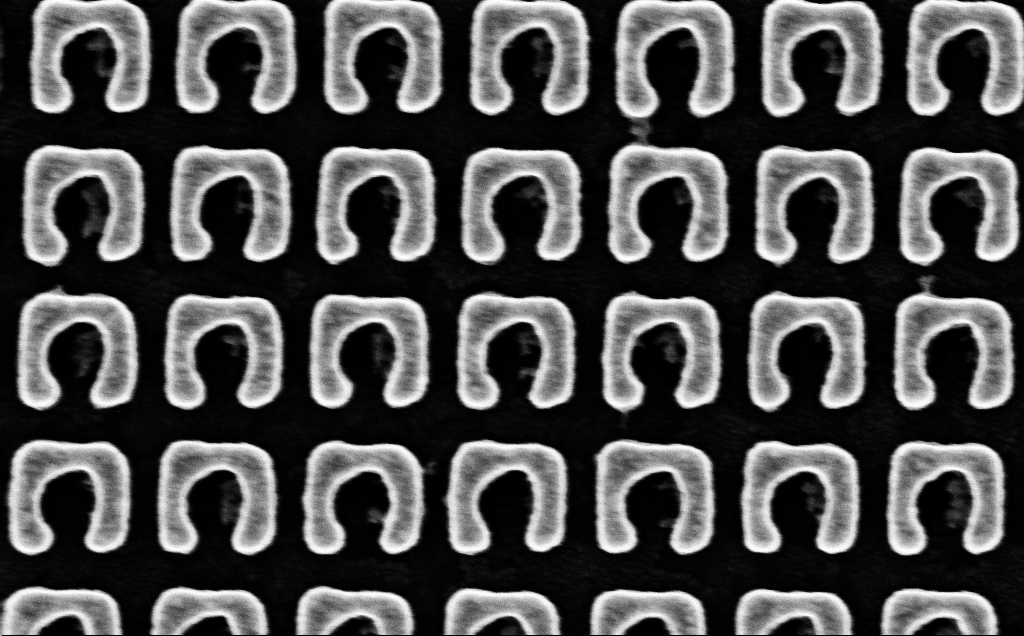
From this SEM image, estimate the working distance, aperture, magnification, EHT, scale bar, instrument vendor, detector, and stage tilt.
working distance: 2.6 mm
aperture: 30 µm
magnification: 116.45 K X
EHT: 5 kV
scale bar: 200 nm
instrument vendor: Zeiss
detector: InLens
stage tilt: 0°